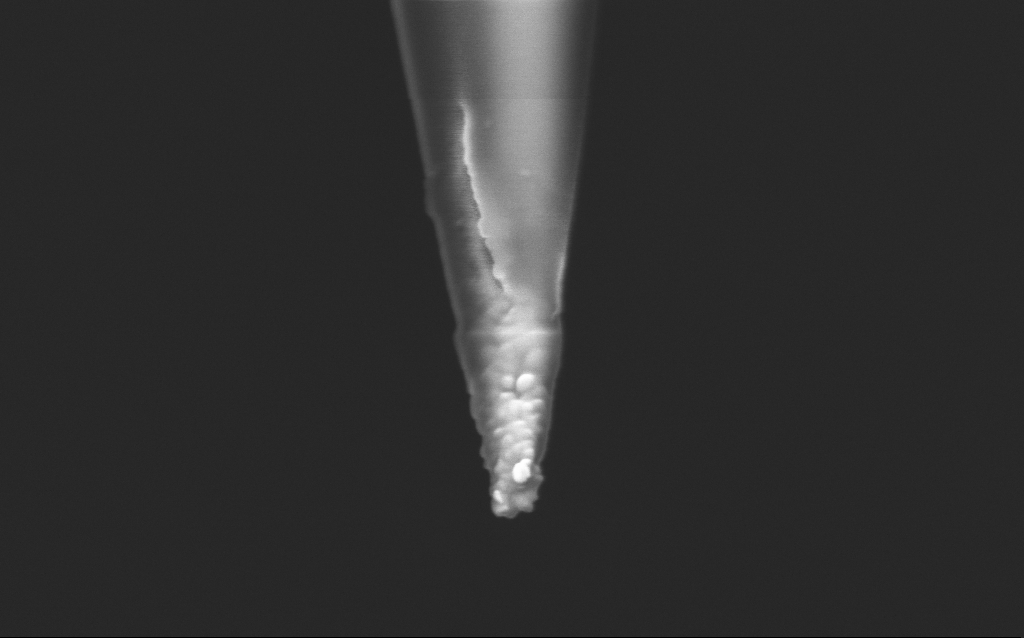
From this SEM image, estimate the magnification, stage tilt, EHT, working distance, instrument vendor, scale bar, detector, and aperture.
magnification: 100 K X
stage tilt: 45°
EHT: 2.5 kV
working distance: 5 mm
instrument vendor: Zeiss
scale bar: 200 nm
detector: InLens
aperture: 30 µm